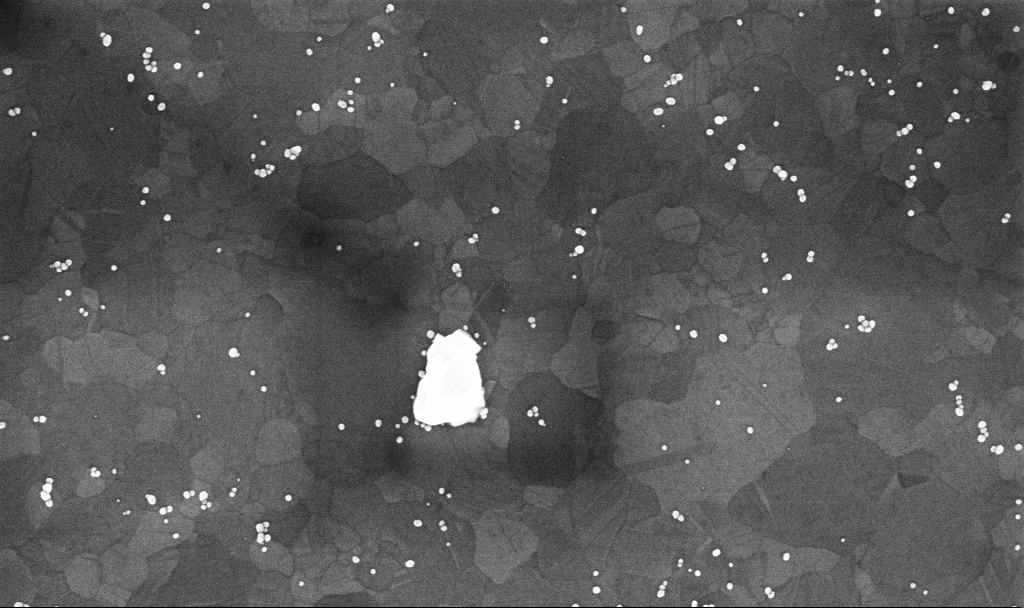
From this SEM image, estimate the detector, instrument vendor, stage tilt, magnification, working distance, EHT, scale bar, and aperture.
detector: InLens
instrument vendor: Zeiss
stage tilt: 0°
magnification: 107.87 K X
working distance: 3.4 mm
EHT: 10 kV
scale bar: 200 nm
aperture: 30 µm